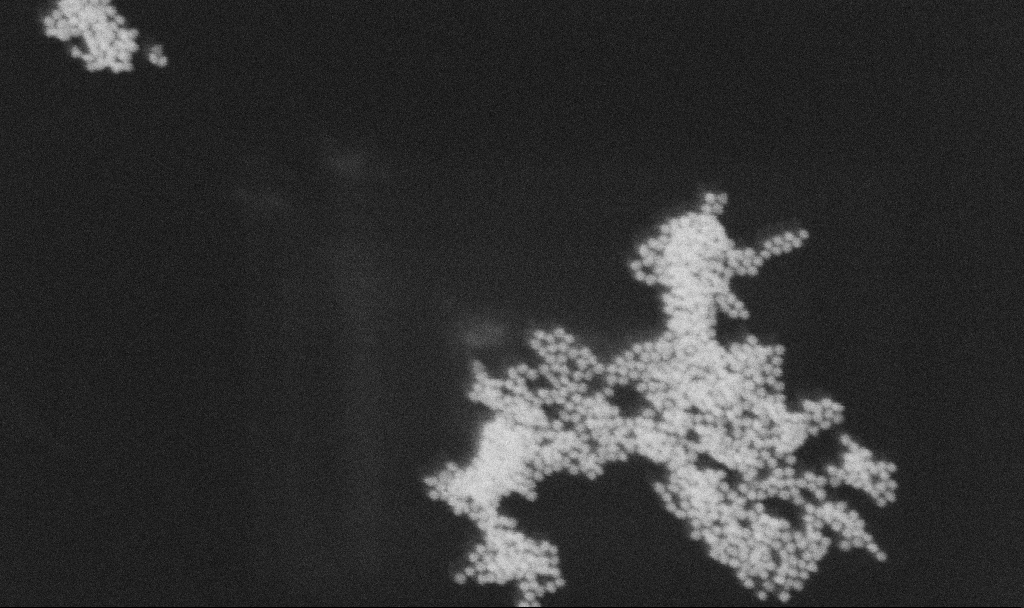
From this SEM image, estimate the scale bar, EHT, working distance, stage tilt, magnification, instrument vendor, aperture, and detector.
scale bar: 200 nm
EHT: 25 kV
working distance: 11.3 mm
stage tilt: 0°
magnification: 205.62 K X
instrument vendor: Zeiss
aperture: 30 µm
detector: SE2